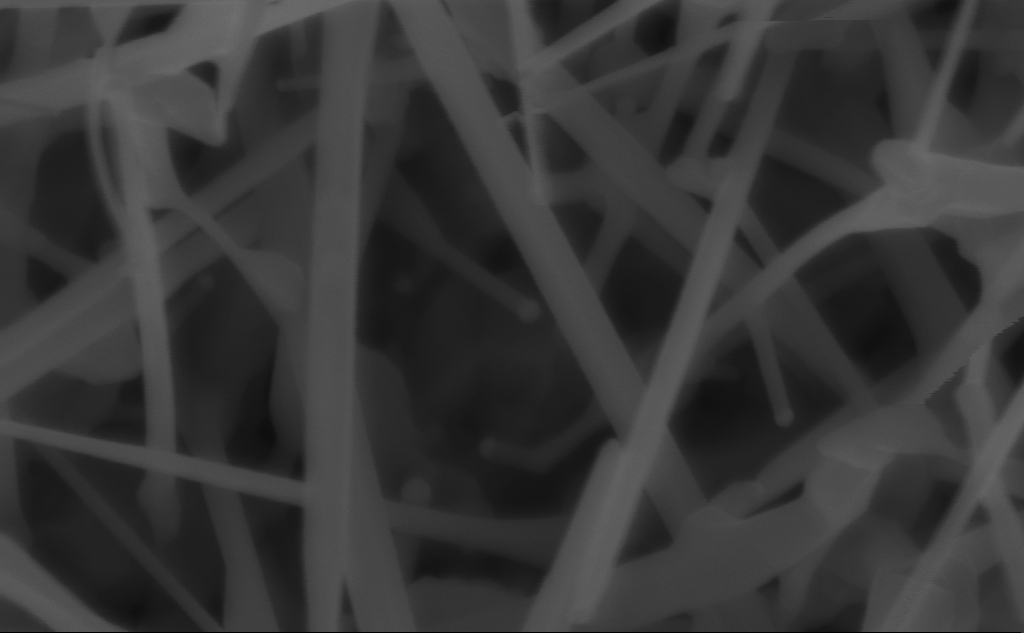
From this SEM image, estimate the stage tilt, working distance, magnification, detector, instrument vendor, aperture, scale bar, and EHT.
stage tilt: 0°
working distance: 4 mm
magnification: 203.93 K X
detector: InLens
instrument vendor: Zeiss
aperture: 30 µm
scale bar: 200 nm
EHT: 5 kV